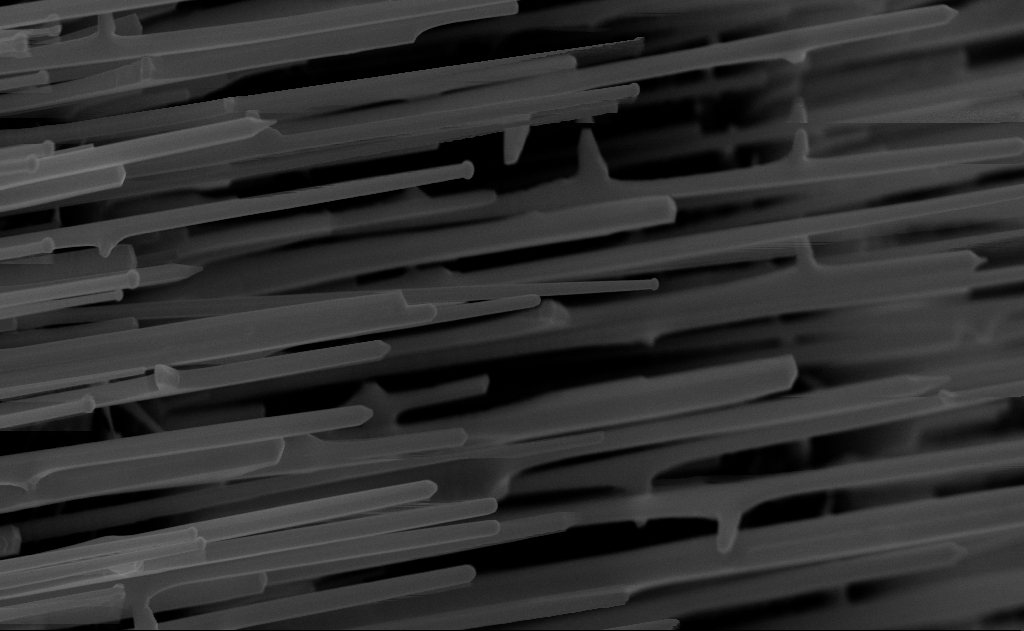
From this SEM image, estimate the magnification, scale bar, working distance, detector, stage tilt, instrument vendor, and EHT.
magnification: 40 K X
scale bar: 1000 nm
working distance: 7 mm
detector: InLens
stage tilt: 0°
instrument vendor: Zeiss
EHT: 10 kV